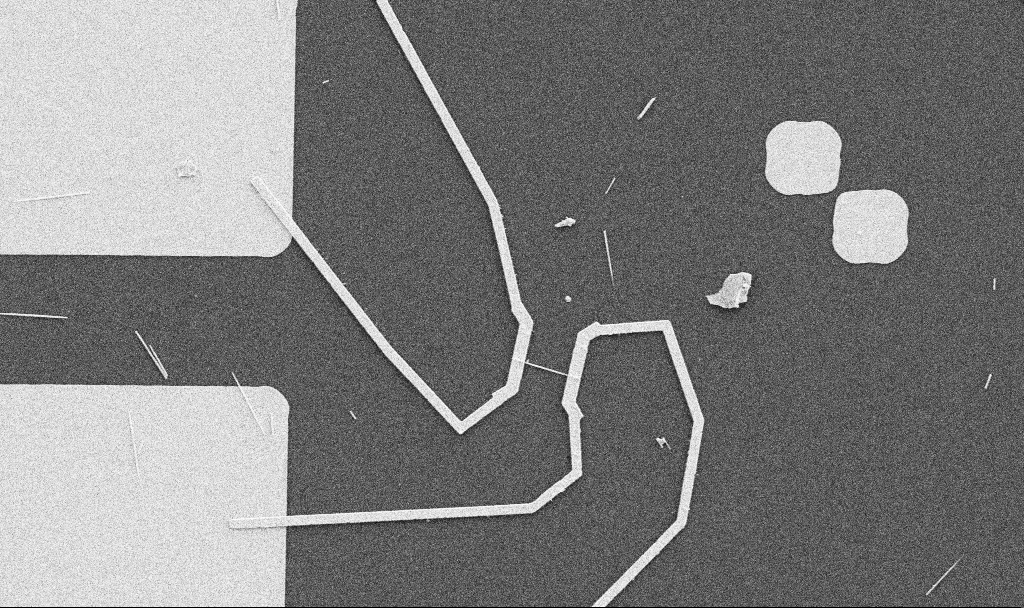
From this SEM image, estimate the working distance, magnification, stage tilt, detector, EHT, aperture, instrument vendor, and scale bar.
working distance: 10.7 mm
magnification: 5 K X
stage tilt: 0°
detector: SE2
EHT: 5 kV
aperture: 30 µm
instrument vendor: Zeiss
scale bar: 10000 nm